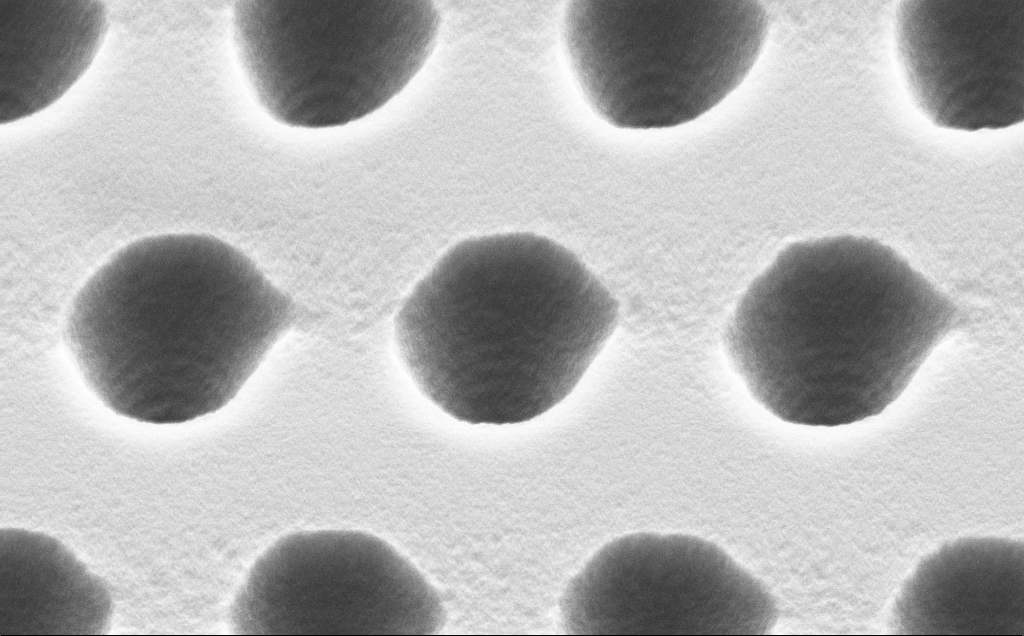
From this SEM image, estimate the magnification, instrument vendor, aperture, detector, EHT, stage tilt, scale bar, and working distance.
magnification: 30.03 K X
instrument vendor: Zeiss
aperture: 30 µm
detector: SE2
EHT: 5 kV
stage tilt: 54°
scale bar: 2000 nm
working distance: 9 mm